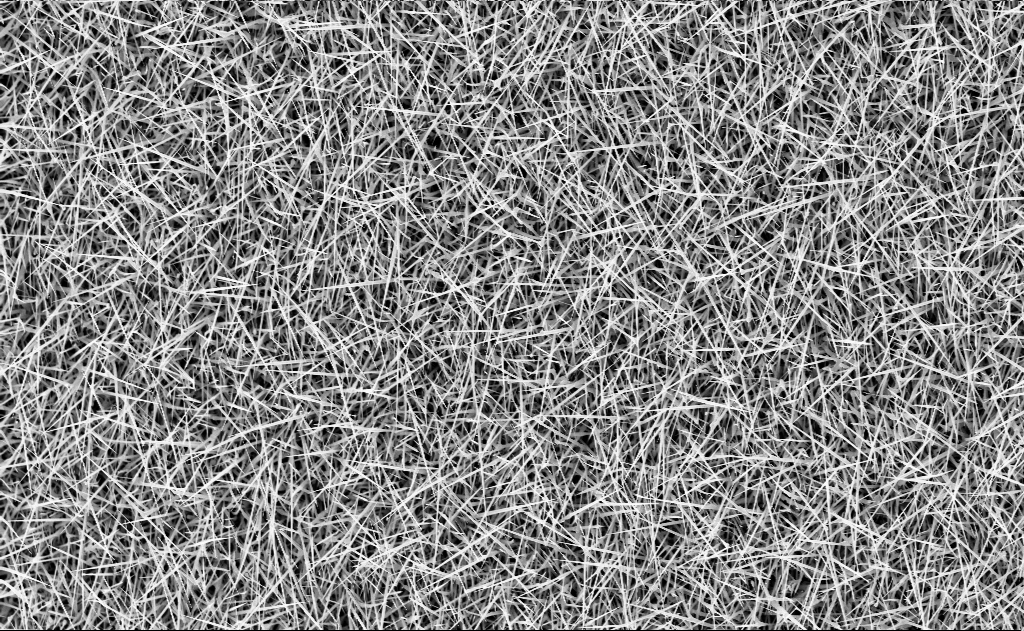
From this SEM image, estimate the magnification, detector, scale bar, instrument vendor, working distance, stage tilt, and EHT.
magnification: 5 K X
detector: InLens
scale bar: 10000 nm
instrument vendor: Zeiss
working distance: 10 mm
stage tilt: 0°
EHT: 10 kV